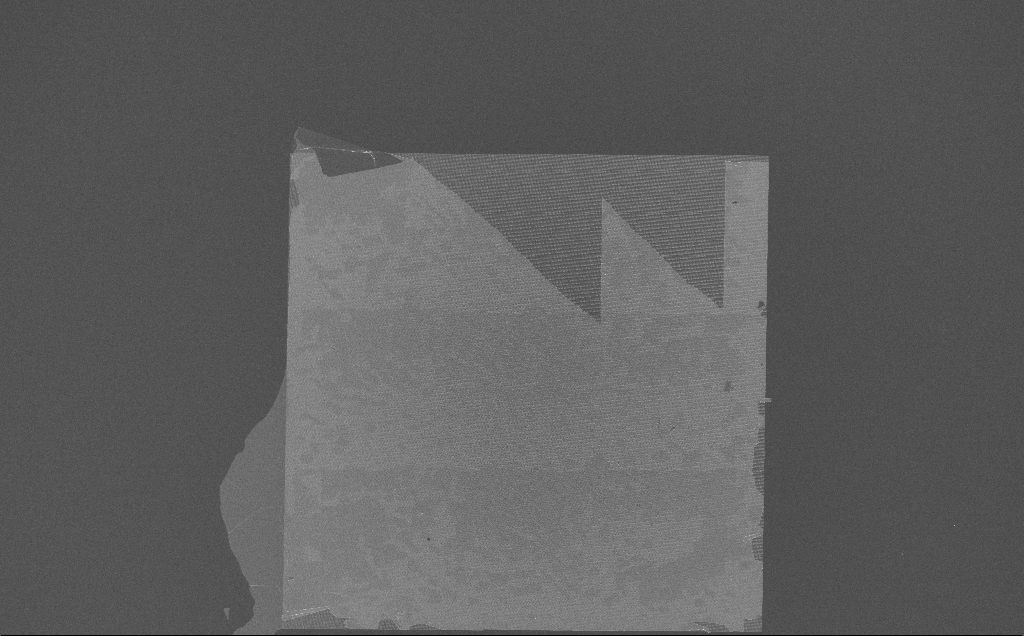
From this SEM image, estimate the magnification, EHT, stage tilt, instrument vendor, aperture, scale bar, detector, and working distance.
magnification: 0.226 K X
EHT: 10 kV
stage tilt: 0°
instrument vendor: Zeiss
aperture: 30 µm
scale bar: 100000 nm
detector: InLens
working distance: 7 mm